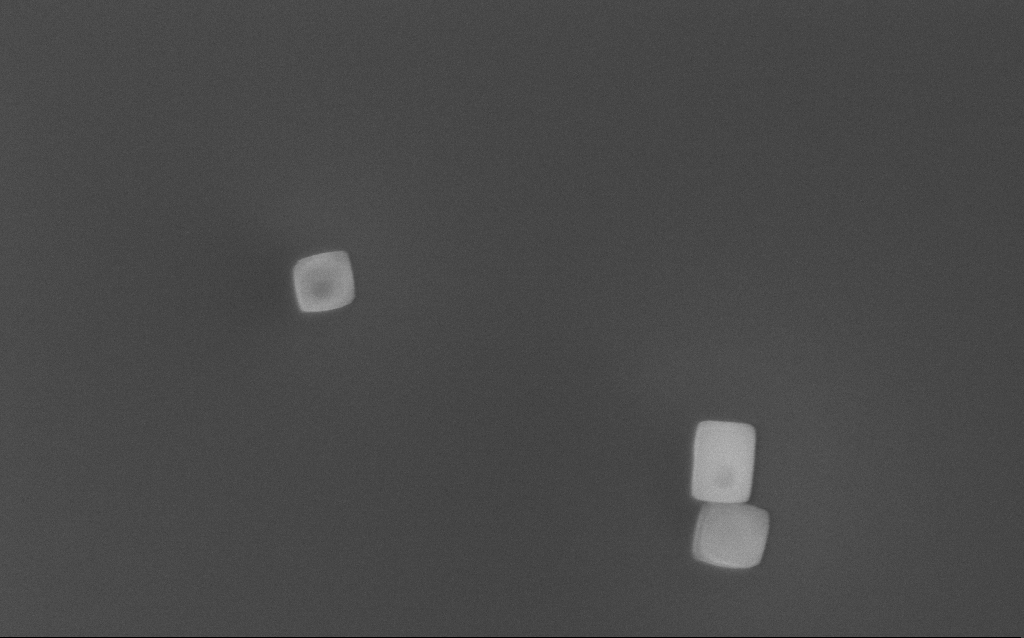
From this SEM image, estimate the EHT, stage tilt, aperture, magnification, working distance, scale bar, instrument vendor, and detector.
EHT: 10 kV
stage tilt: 0°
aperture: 30 µm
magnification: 94.03 K X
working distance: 3 mm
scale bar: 200 nm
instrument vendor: Zeiss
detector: InLens